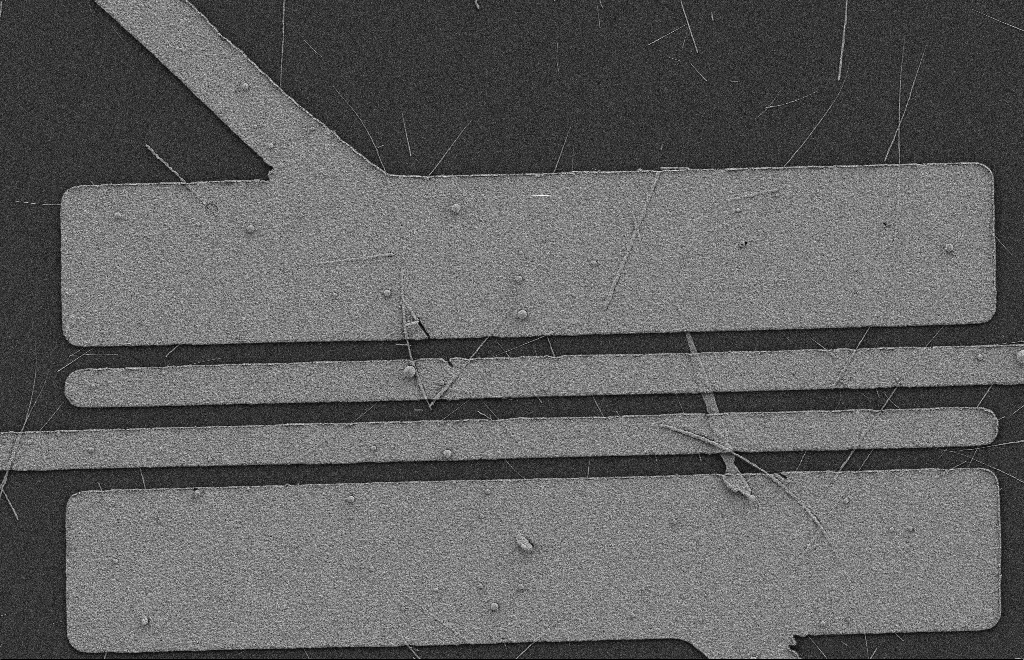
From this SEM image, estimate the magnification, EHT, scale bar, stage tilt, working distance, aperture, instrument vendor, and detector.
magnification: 5.61 K X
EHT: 2 kV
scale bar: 2000 nm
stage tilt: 0°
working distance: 9 mm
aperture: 20 µm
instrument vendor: Zeiss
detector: SE2